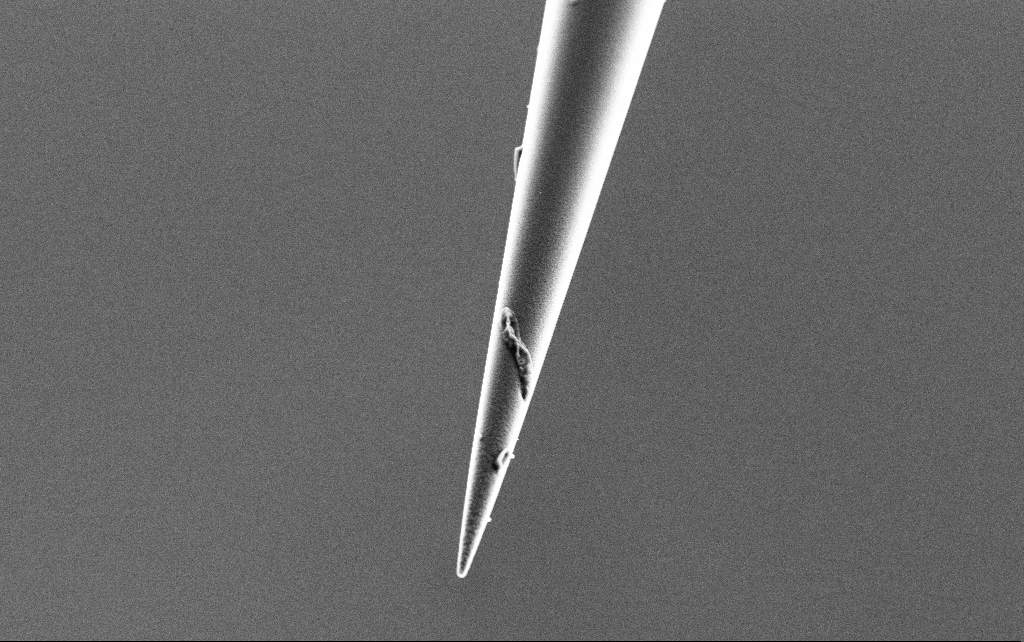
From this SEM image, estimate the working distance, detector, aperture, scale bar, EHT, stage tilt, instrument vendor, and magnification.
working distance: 7.4 mm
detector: SE2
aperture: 30 µm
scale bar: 2000 nm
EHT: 3 kV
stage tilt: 45°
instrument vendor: Zeiss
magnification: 10 K X